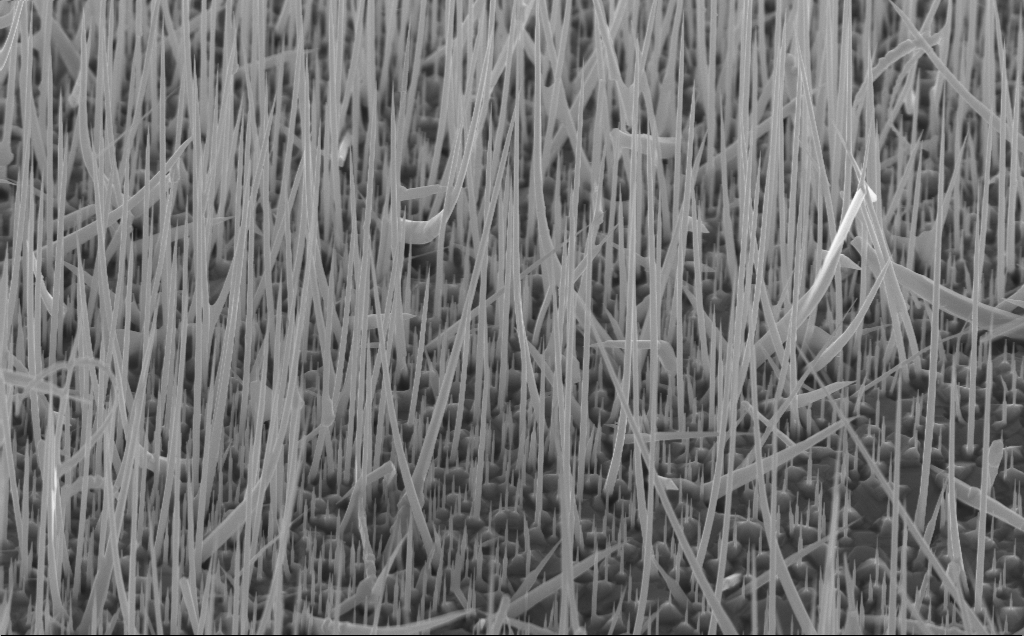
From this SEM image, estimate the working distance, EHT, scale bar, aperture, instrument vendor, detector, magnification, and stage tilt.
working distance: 4 mm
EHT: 10 kV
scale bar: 1000 nm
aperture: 30 µm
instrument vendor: Zeiss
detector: InLens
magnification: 18.6 K X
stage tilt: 45°